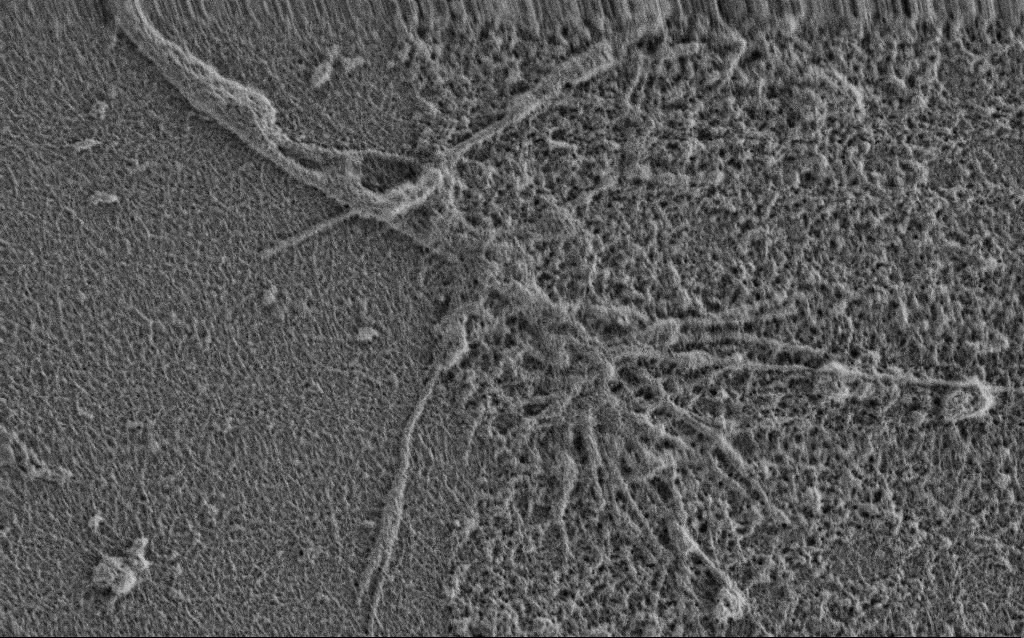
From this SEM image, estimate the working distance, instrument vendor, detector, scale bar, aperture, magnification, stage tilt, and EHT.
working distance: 3.4 mm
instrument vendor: Zeiss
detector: SE2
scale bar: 2000 nm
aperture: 30 µm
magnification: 15 K X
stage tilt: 0°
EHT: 0.9 kV